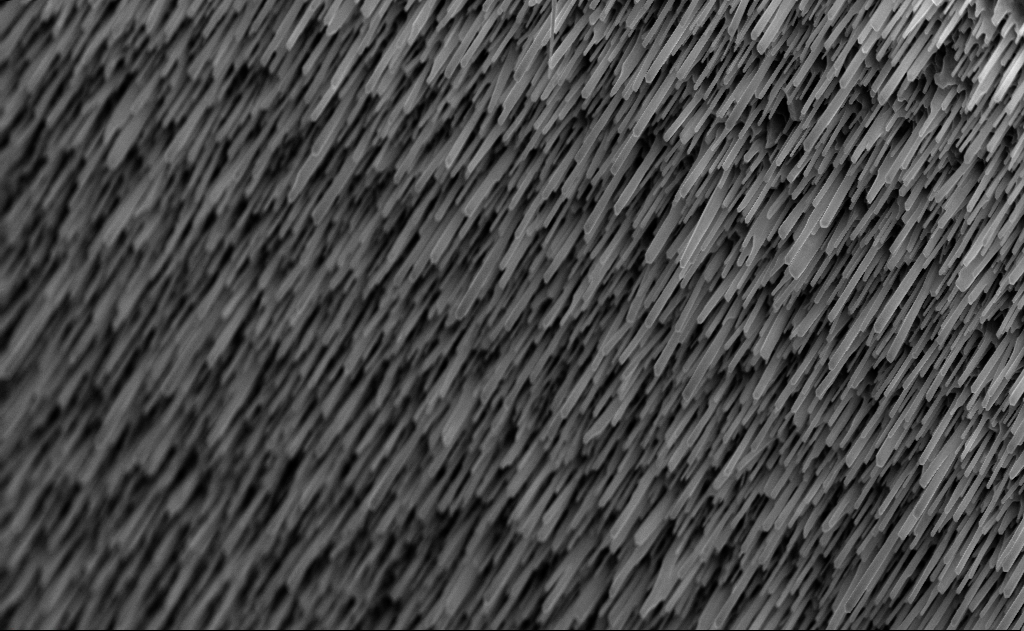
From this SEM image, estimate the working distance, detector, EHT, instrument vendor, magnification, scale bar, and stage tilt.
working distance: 7 mm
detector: InLens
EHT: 10 kV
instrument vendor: Zeiss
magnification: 20 K X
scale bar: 2000 nm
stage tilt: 0°